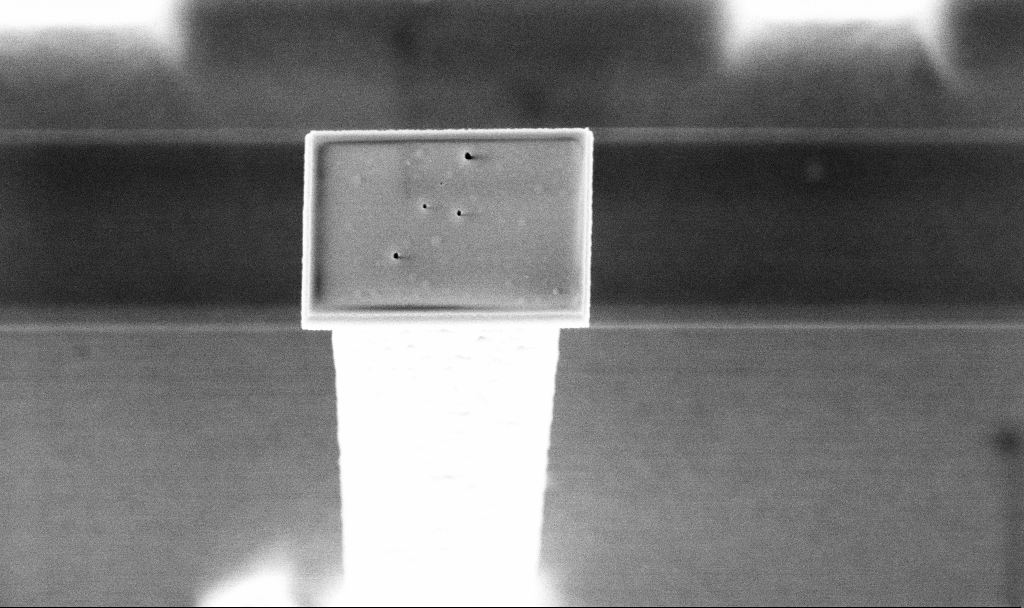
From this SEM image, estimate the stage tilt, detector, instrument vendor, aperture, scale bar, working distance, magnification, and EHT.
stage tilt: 20°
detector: InLens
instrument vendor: Zeiss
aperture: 30 µm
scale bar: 1000 nm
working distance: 4.7 mm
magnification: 24.05 K X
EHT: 5 kV